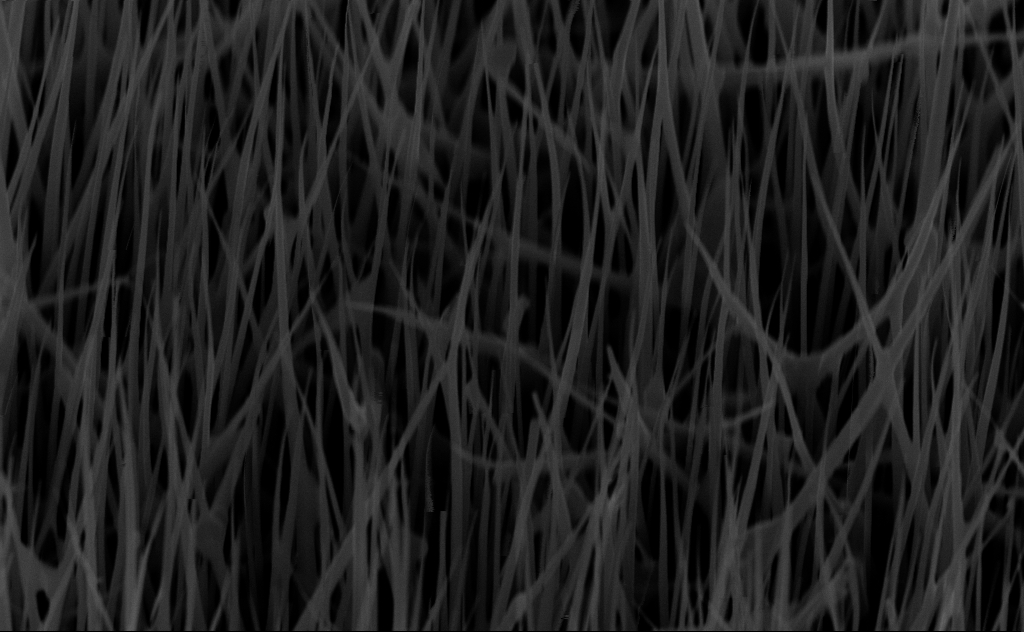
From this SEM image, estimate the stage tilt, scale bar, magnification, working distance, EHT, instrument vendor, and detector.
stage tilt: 45°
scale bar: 1000 nm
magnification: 40 K X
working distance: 6 mm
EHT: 10 kV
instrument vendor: Zeiss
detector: InLens